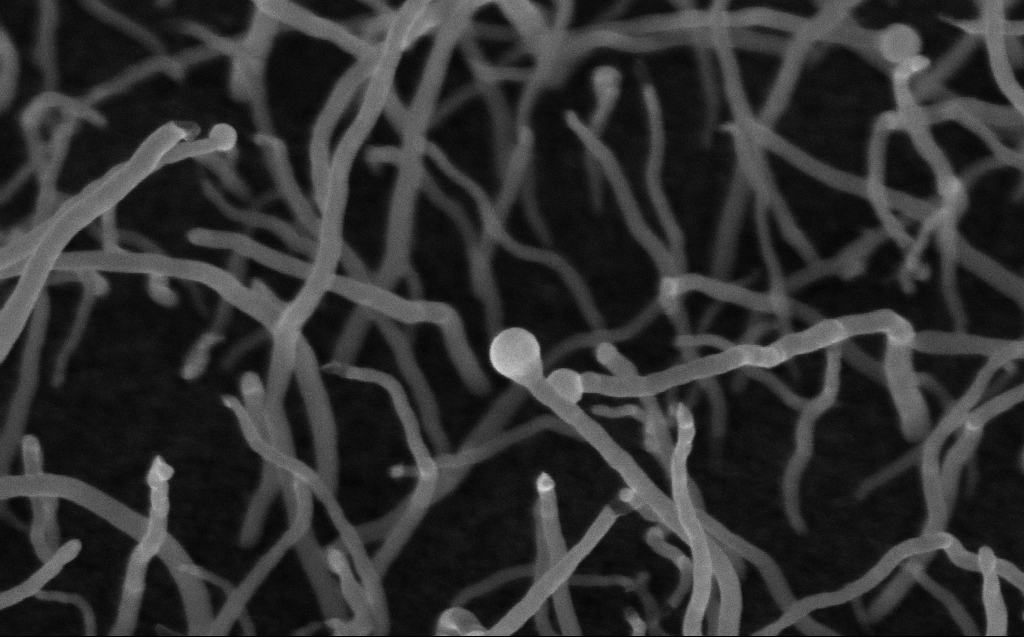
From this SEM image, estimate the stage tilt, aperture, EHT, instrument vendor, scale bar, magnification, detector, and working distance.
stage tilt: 45°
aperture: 30 µm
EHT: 10 kV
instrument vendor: Zeiss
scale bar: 200 nm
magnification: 144.5 K X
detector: InLens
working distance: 6 mm